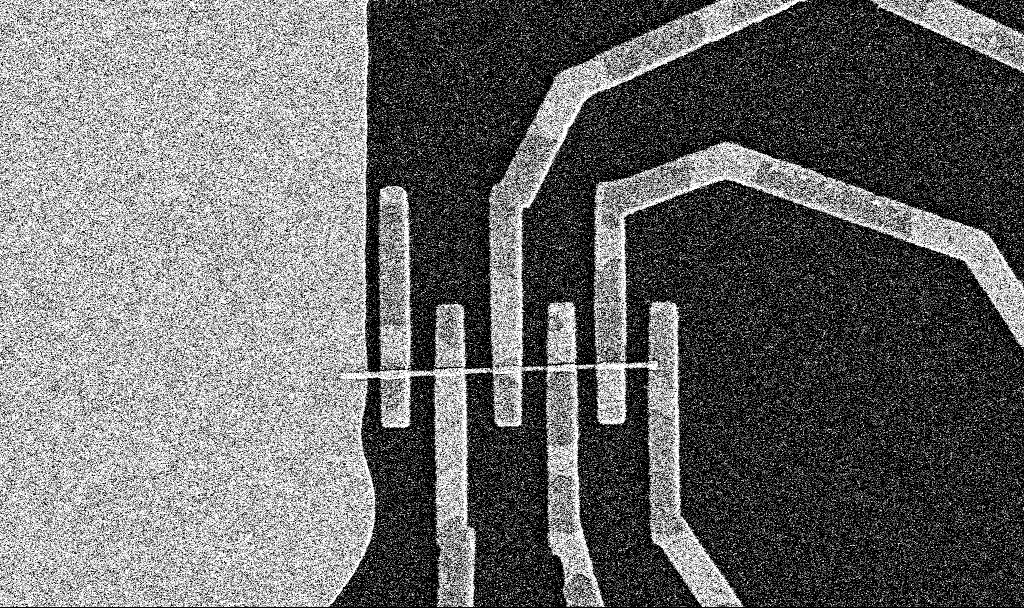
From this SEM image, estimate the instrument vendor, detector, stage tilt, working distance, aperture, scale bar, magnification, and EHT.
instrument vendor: Zeiss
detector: SE2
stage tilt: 0°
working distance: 8.5 mm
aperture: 30 µm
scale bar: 2000 nm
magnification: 17.55 K X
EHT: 5 kV